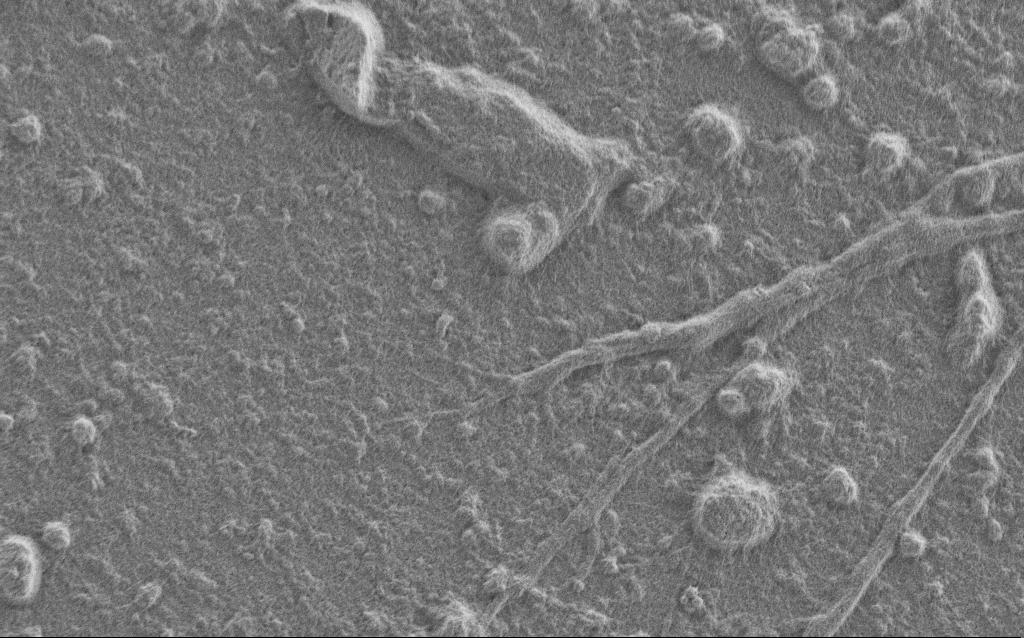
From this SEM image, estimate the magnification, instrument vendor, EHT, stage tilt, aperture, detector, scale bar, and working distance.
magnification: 7.5 K X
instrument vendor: Zeiss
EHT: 1 kV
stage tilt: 0°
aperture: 30 µm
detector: SE2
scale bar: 2000 nm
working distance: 6 mm